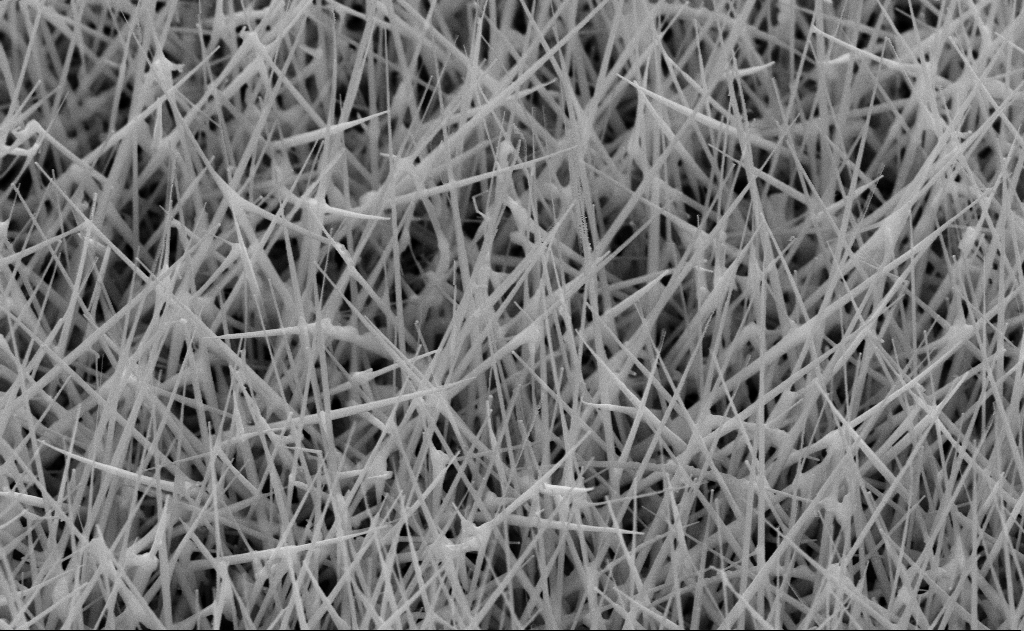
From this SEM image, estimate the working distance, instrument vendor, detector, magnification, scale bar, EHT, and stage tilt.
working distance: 11 mm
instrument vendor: Zeiss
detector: SE2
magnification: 40 K X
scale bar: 1000 nm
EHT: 10 kV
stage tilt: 45°